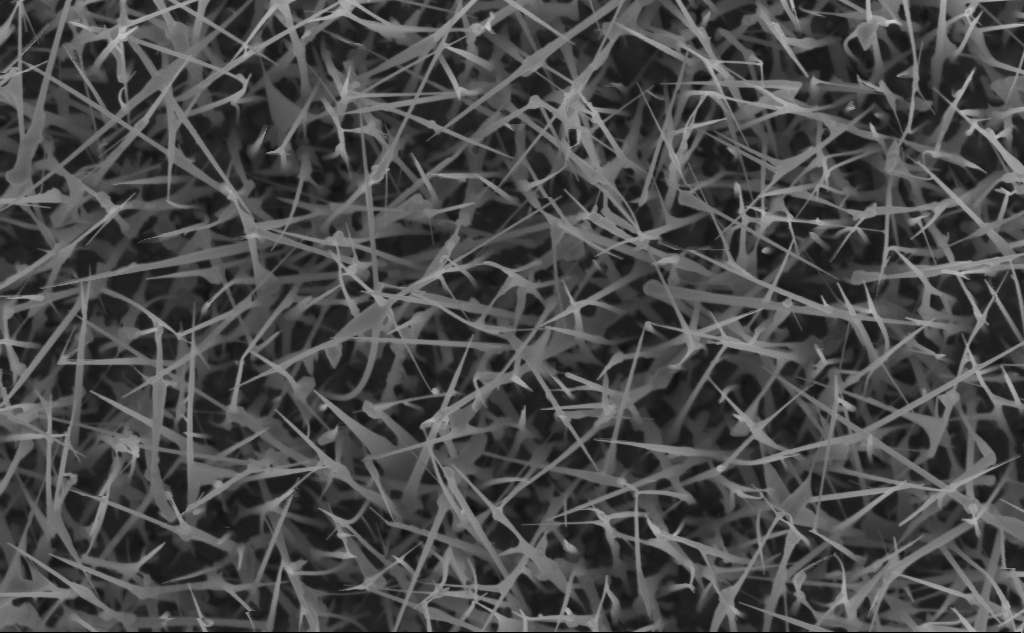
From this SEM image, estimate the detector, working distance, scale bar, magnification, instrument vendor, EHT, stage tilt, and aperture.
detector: InLens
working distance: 6 mm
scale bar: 1000 nm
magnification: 40 K X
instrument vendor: Zeiss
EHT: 5 kV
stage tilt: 0°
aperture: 30 µm